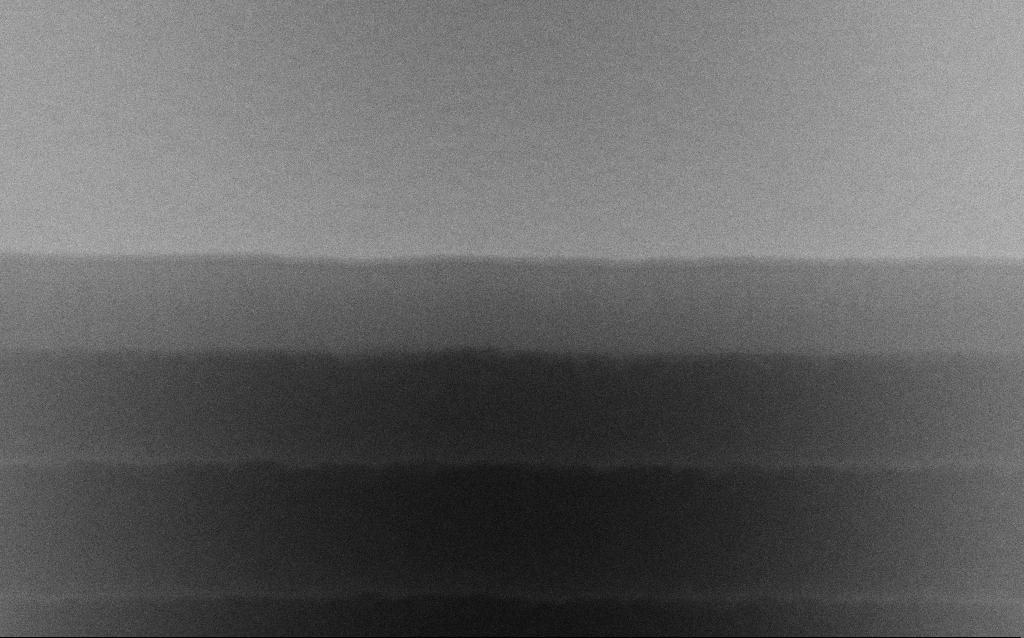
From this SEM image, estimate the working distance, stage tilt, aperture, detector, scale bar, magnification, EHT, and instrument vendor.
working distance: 4.8 mm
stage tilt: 45°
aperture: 30 µm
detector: SE2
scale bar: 100 nm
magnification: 239.8 K X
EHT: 10 kV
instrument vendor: Zeiss